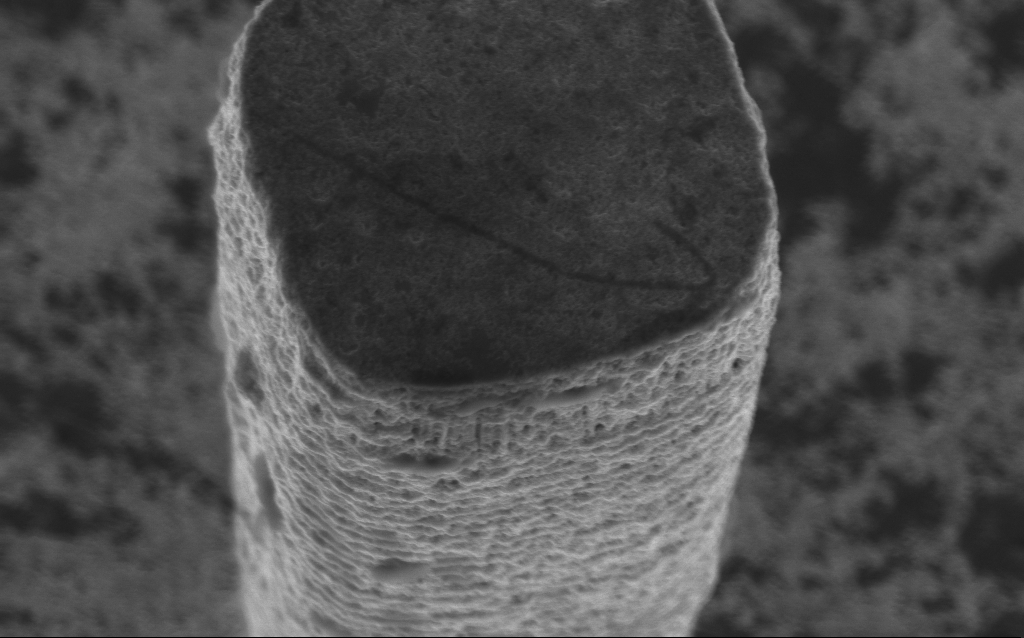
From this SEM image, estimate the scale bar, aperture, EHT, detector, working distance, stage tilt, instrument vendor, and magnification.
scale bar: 1000 nm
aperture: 30 µm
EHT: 5 kV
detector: InLens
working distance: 5 mm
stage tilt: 23.7°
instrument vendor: Zeiss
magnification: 30.09 K X